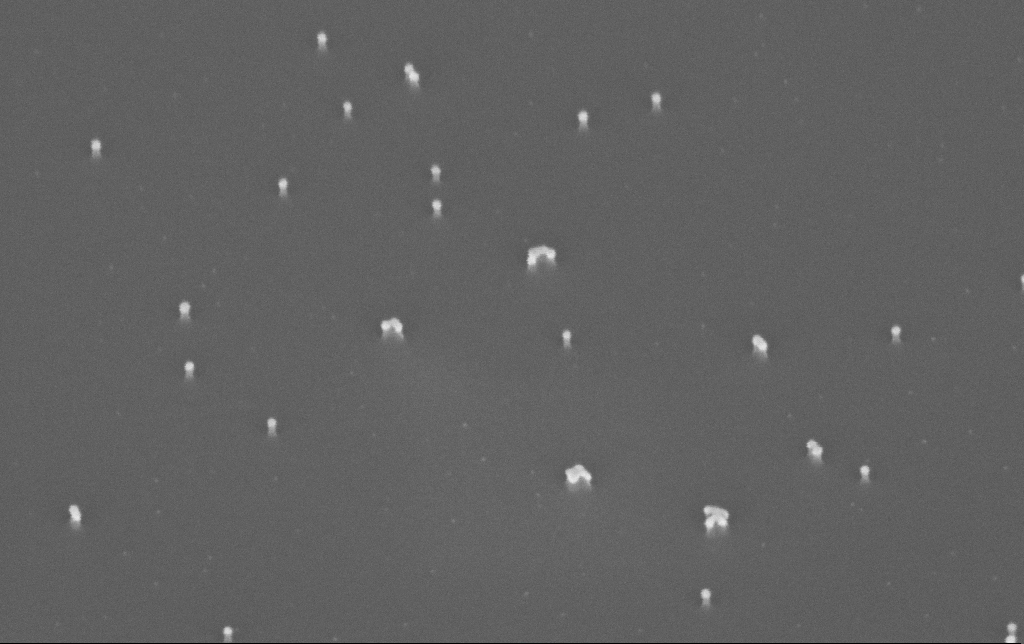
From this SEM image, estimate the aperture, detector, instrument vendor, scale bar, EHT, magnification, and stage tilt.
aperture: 30 µm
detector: InLens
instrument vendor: Zeiss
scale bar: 200 nm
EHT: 10 kV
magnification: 200 K X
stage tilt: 45°